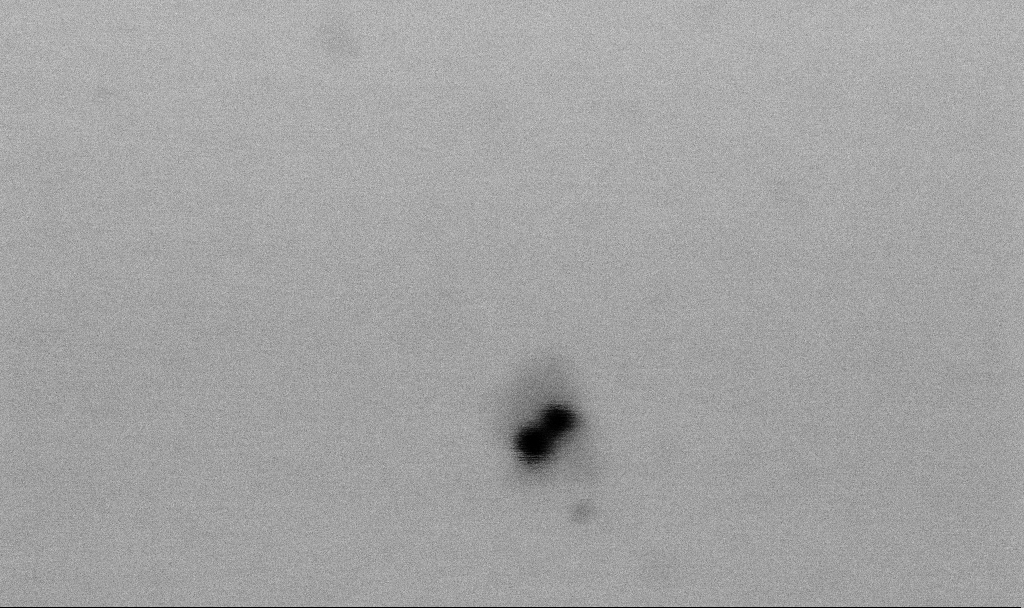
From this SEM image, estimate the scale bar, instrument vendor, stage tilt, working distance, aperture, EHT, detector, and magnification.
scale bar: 100 nm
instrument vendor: Zeiss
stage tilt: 0°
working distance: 6.5 mm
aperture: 30 µm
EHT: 2 kV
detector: SE2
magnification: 400 K X